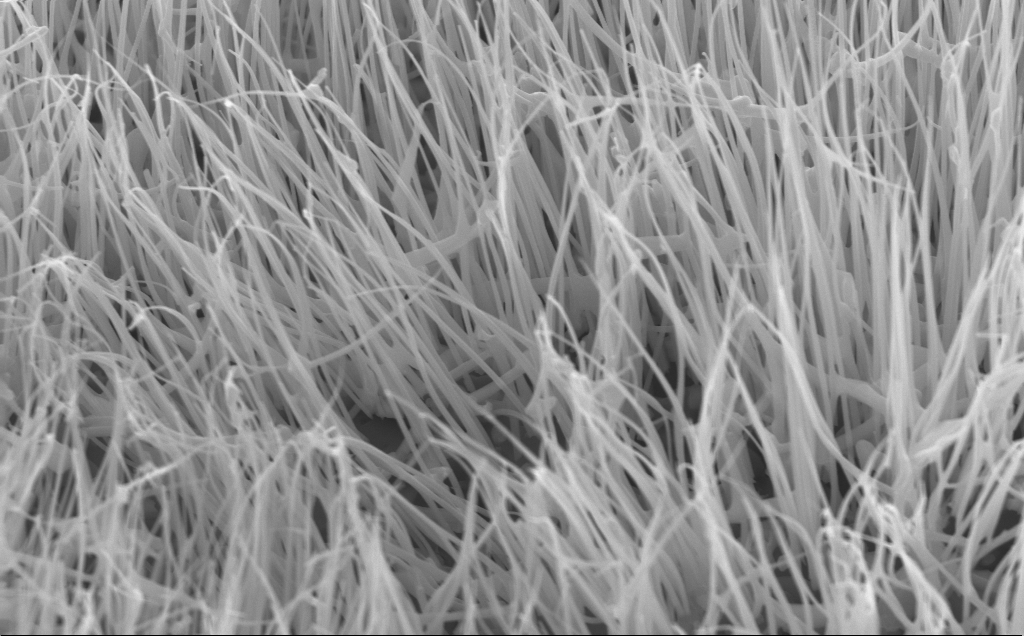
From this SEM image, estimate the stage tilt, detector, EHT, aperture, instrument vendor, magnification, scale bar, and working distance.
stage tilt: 45°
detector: InLens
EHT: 10 kV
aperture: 30 µm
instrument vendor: Zeiss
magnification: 40 K X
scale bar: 1000 nm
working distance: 6 mm